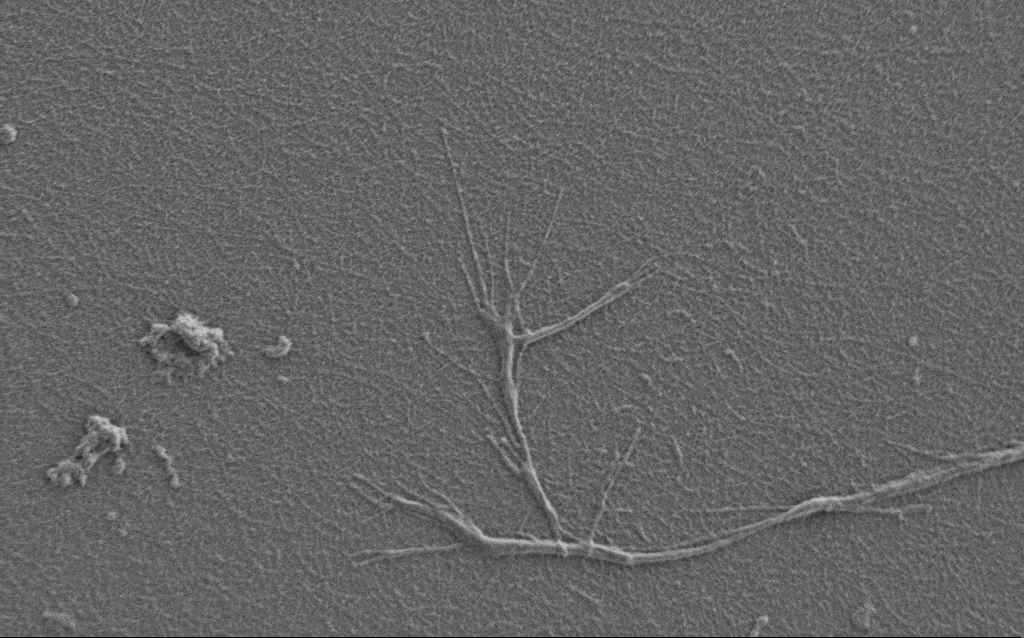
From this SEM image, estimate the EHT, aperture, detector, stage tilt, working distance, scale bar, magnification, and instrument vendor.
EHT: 0.9 kV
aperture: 30 µm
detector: SE2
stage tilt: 0°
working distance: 7 mm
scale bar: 2000 nm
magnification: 10 K X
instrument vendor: Zeiss